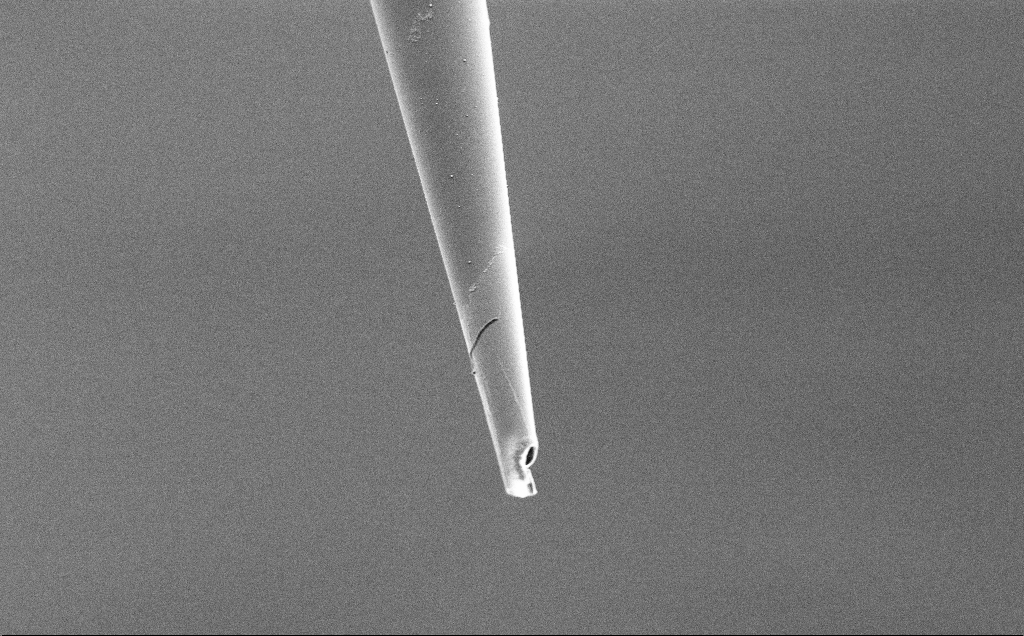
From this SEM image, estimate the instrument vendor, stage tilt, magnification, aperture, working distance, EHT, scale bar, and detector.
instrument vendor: Zeiss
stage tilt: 45°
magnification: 3 K X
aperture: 30 µm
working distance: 7.8 mm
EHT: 3 kV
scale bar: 20000 nm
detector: SE2